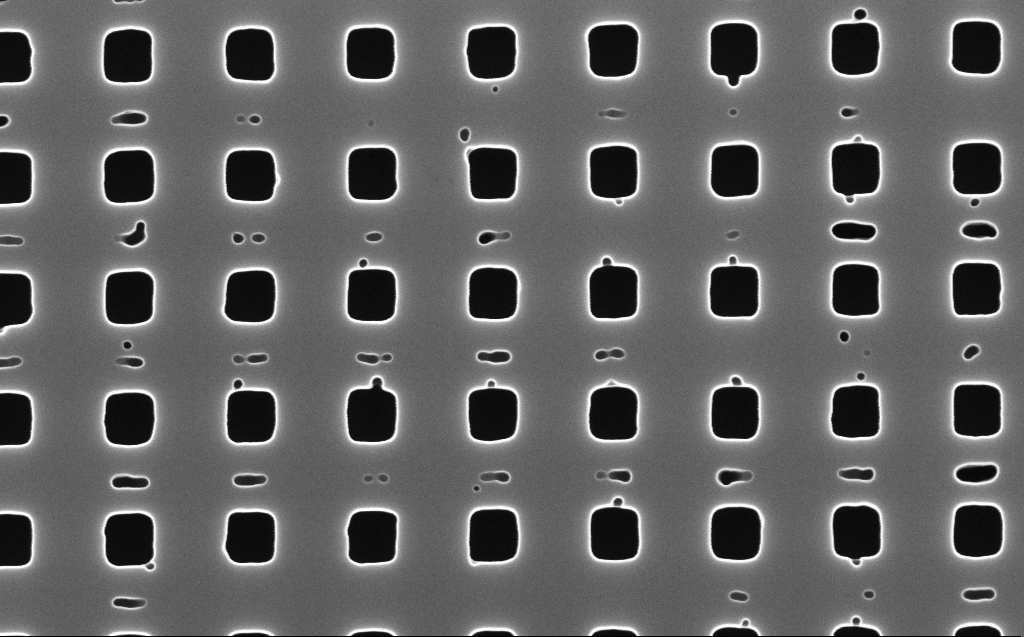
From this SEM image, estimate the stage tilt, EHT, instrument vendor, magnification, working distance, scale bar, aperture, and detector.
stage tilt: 0°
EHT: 10 kV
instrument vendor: Zeiss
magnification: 90 K X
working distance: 5 mm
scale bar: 200 nm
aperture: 30 µm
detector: InLens